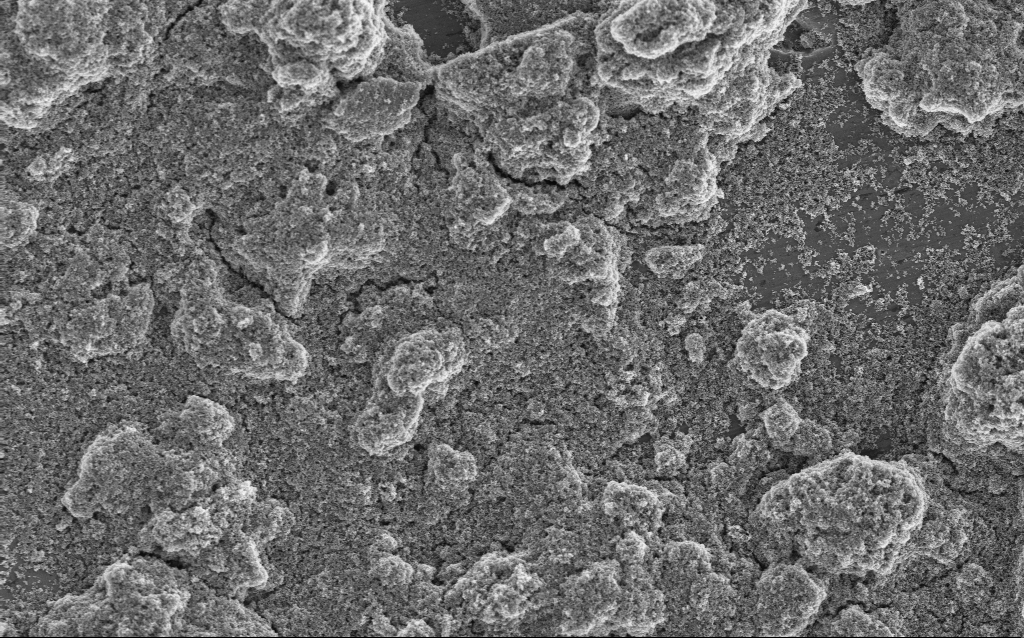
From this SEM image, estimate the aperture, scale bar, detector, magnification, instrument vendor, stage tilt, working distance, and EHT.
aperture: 30 µm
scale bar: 10000 nm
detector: InLens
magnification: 6.41 K X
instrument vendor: Zeiss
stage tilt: -0°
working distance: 4.2 mm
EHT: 5 kV